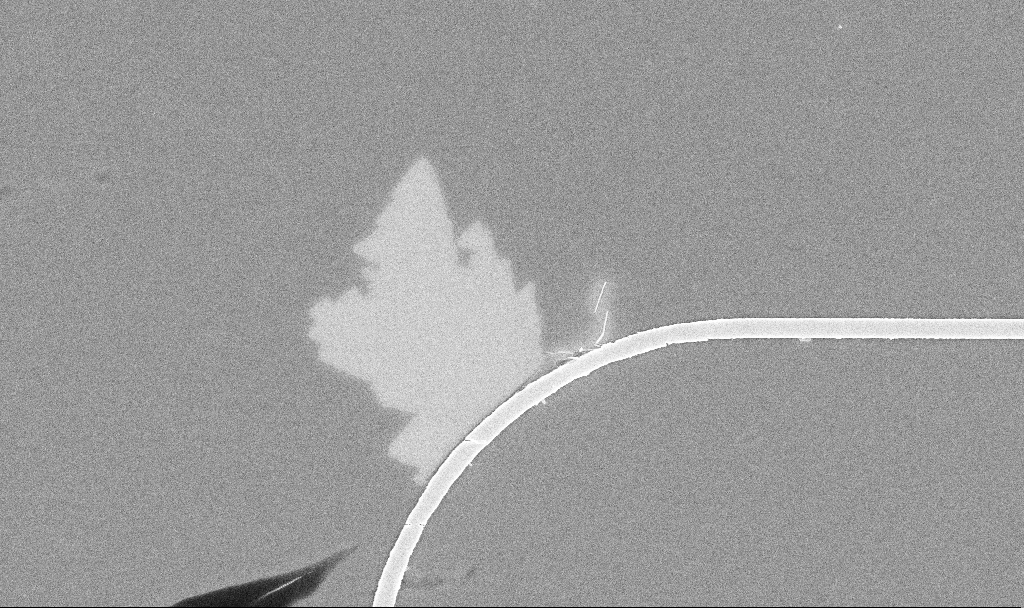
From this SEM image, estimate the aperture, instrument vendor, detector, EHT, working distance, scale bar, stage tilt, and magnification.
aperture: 30 µm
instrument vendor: Zeiss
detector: InLens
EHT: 5 kV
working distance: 10.3 mm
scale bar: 2000 nm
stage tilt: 0°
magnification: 13.96 K X